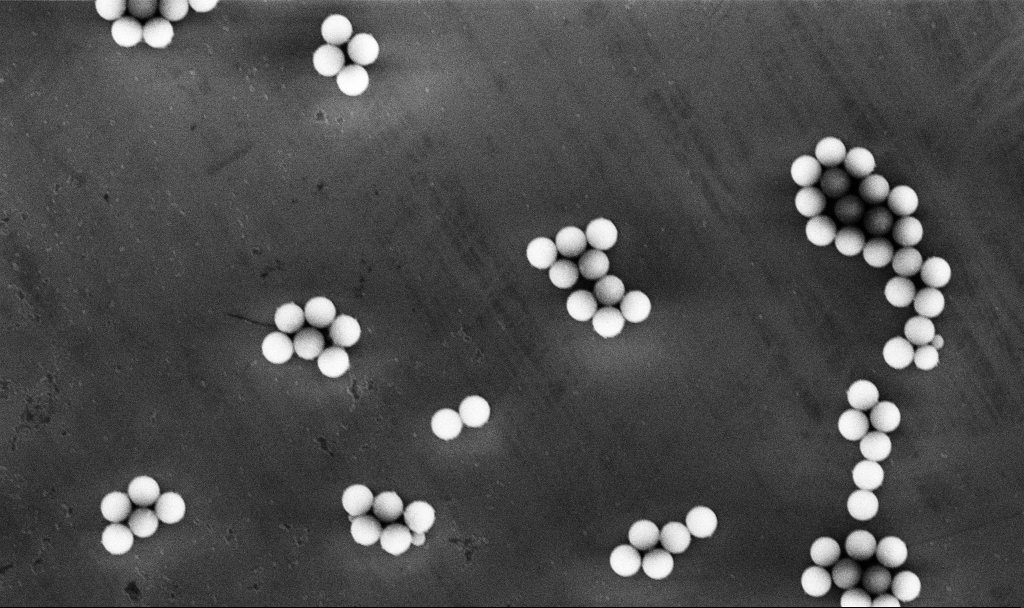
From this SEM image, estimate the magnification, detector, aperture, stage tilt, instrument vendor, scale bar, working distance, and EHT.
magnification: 5.42 K X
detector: InLens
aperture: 30 µm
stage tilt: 0°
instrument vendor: Zeiss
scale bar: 10000 nm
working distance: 5.2 mm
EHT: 10 kV